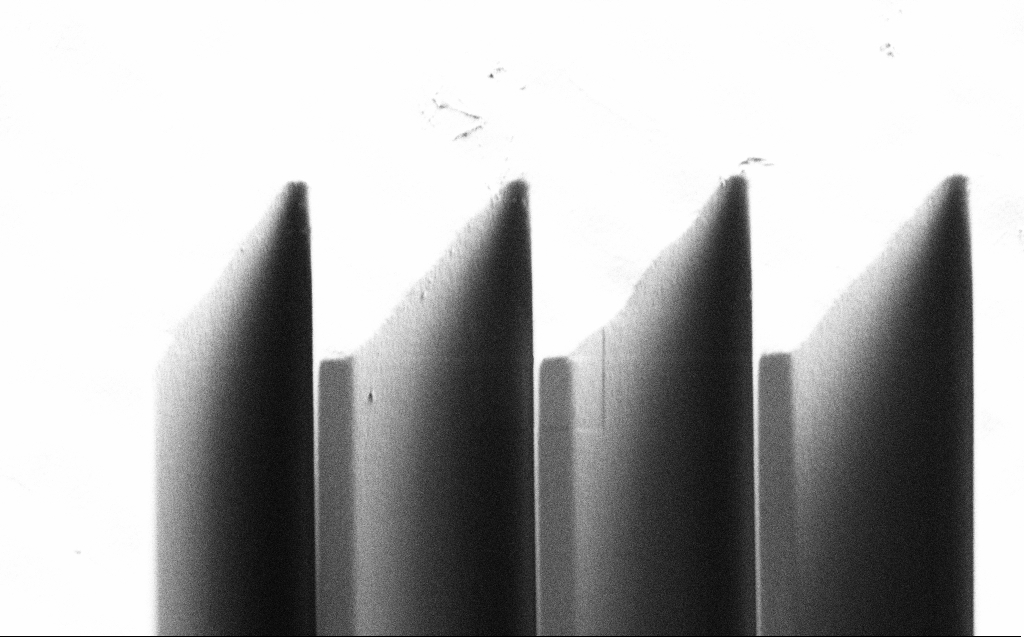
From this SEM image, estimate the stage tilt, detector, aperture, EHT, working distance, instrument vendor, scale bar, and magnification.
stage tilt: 45°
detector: SE2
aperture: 30 µm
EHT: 1 kV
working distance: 7 mm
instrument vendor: Zeiss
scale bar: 10000 nm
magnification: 7 K X